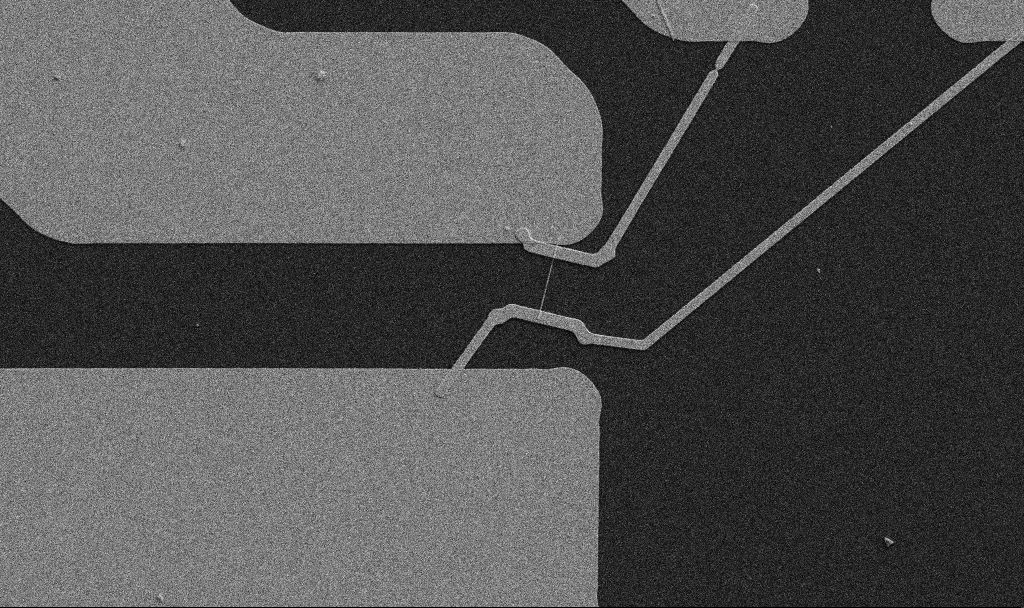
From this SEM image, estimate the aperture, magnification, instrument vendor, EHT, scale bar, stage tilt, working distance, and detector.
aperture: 30 µm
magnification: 5 K X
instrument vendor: Zeiss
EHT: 5 kV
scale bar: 10000 nm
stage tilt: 0°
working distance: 10.7 mm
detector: SE2